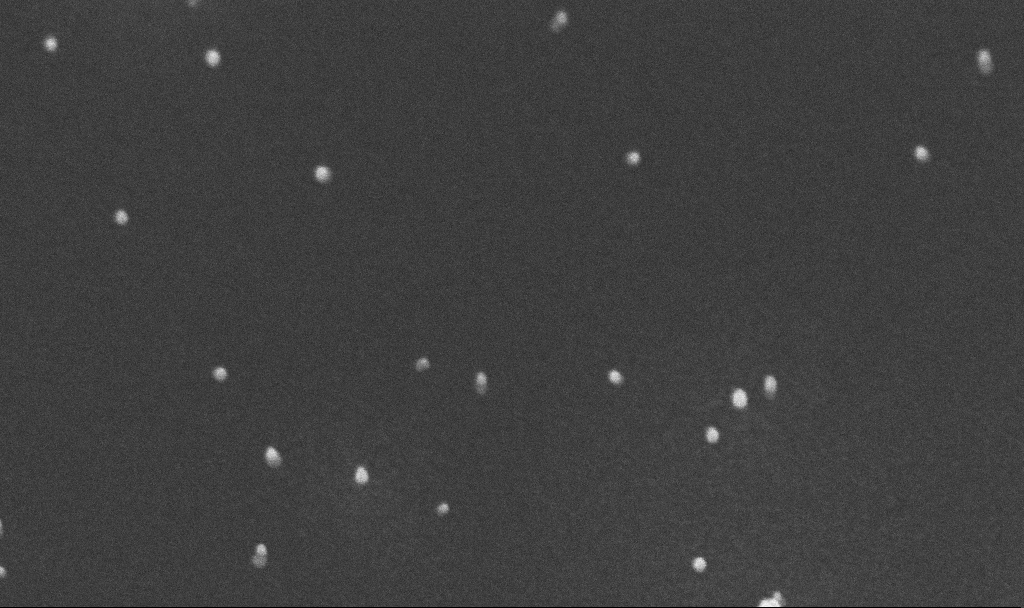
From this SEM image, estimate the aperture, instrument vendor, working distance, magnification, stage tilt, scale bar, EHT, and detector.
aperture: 30 µm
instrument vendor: Zeiss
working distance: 4.8 mm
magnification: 200 K X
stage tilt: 45°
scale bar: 100 nm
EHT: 10 kV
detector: InLens